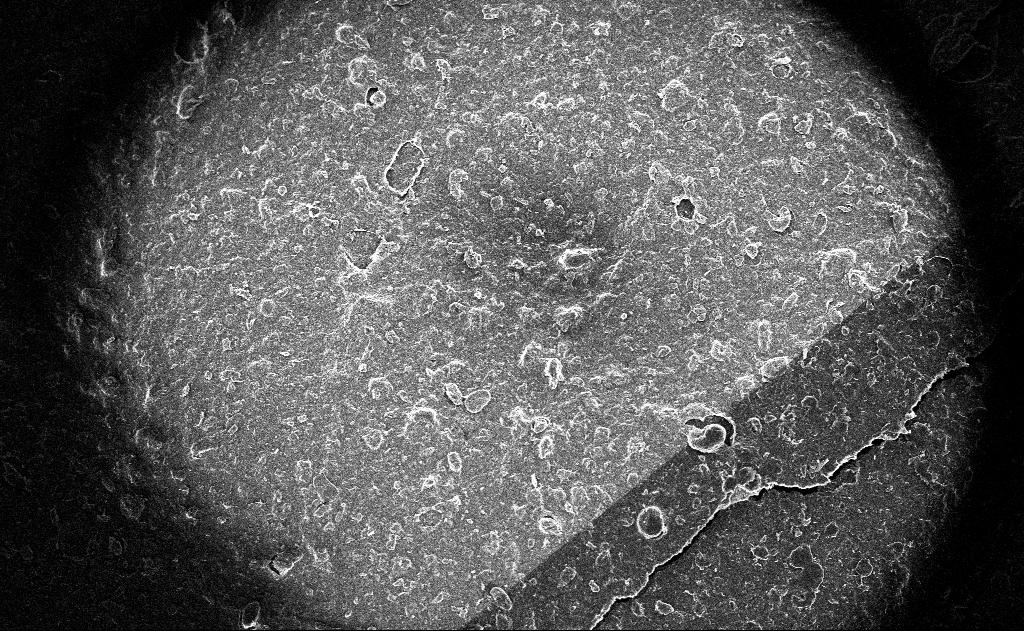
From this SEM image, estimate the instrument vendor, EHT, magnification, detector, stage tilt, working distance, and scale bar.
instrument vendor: Zeiss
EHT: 10 kV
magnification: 0.119 K X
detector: InLens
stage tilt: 0°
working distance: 3 mm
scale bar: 200000 nm